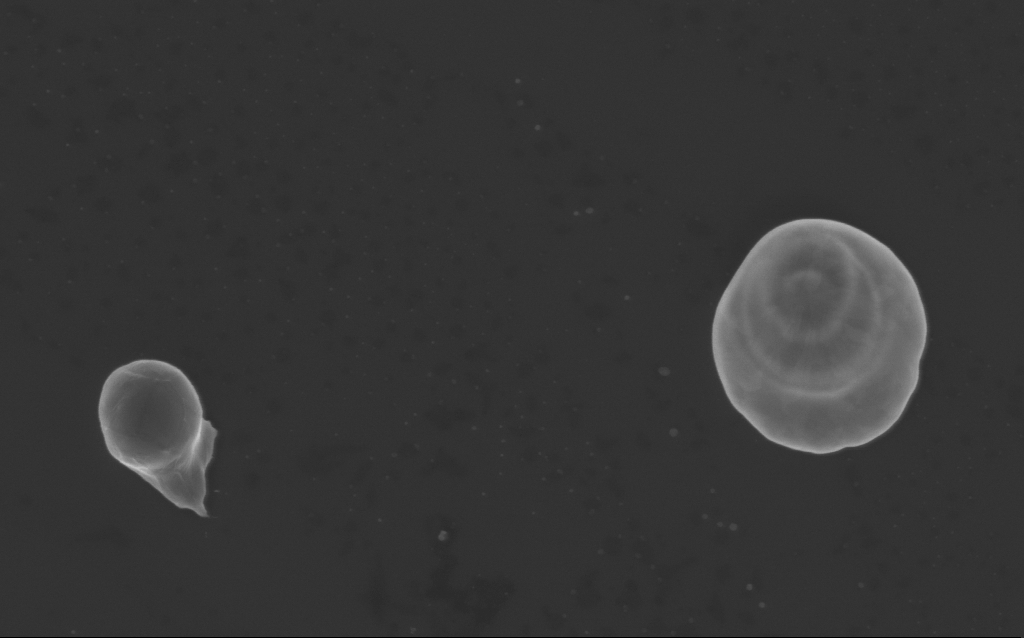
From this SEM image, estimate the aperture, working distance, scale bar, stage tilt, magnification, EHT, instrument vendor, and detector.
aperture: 30 µm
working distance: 4 mm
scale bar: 1000 nm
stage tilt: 0°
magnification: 48.29 K X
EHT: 3 kV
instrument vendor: Zeiss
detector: InLens